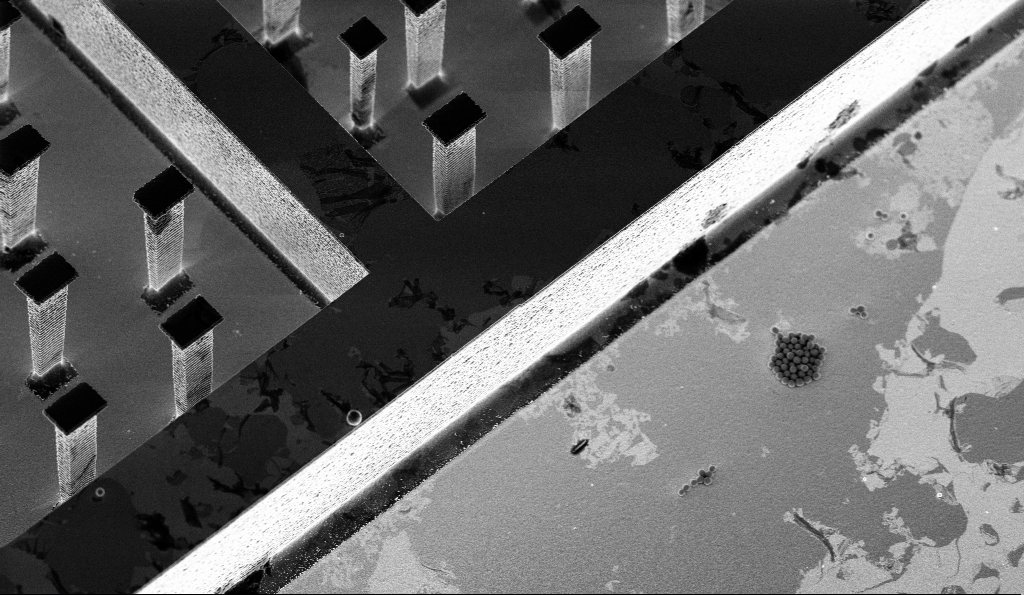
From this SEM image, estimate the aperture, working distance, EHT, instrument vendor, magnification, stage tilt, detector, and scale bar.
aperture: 30 µm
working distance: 5.5 mm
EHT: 3 kV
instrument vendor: Zeiss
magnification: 4.62 K X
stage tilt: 30°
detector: InLens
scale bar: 10000 nm